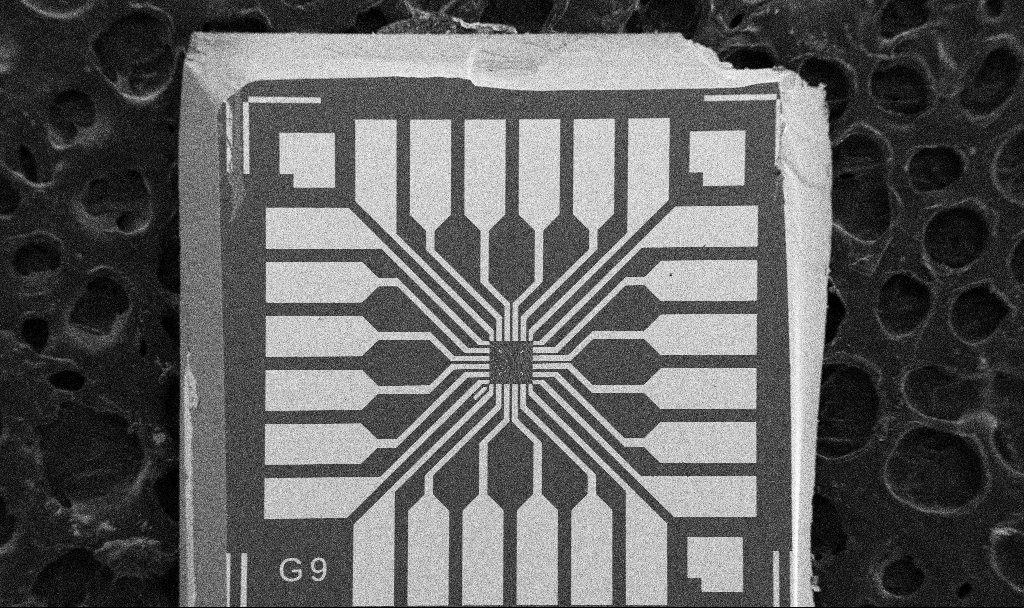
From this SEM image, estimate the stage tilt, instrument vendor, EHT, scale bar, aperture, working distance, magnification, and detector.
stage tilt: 0°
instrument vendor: Zeiss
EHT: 5 kV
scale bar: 200000 nm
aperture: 30 µm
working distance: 10.6 mm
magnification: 0.1 K X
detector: SE2